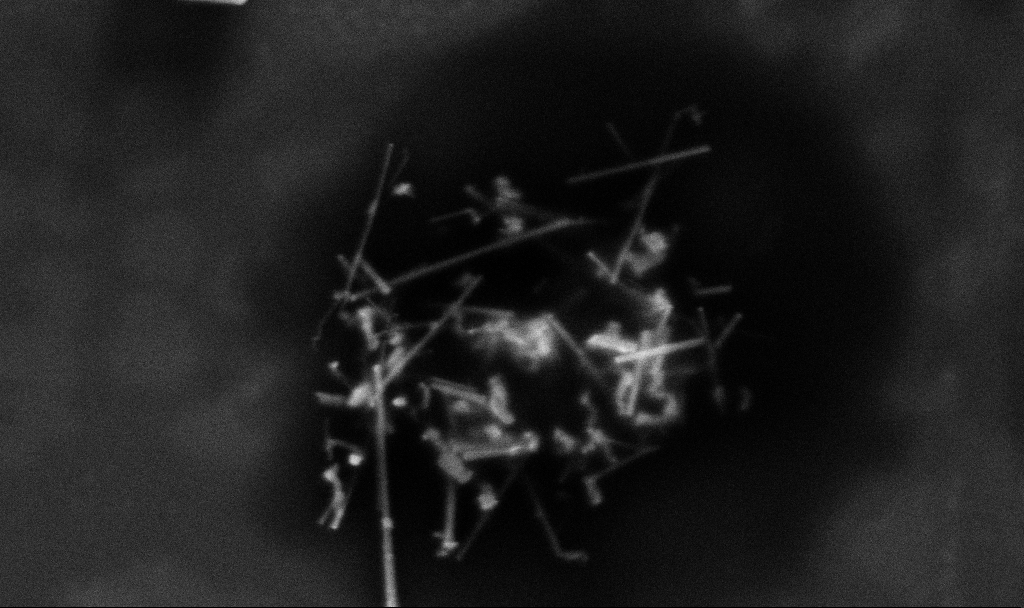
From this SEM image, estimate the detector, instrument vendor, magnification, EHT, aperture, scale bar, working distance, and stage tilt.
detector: InLens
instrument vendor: Zeiss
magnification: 69.41 K X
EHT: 3 kV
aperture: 30 µm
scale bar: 1000 nm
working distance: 3.3 mm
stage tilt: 0°